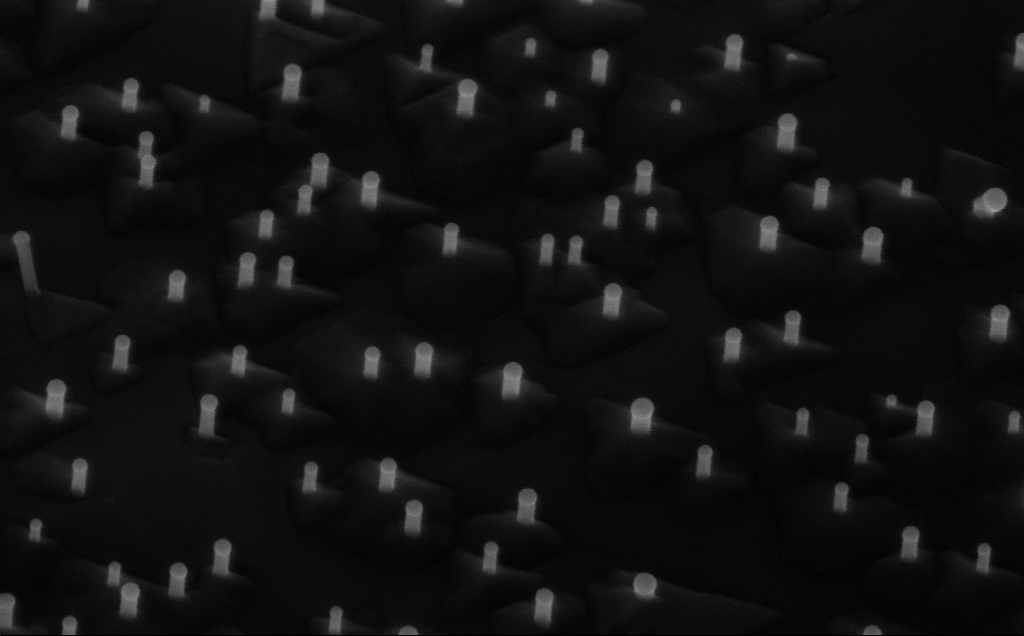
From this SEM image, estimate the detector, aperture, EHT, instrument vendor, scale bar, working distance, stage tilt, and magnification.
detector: InLens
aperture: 30 µm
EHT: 10 kV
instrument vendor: Zeiss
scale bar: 100 nm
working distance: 6 mm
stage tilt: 45°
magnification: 150 K X